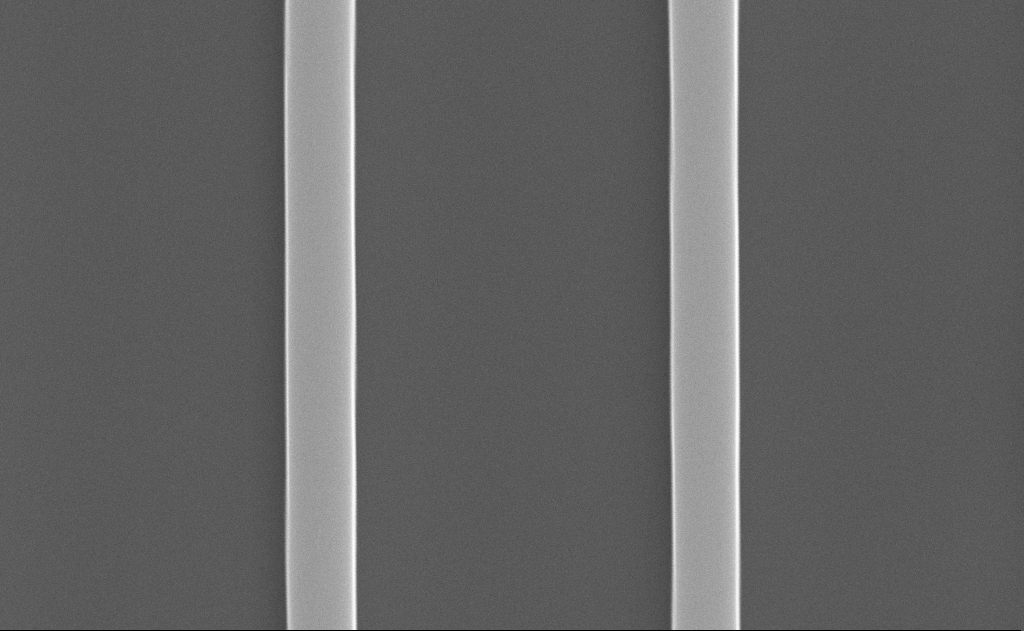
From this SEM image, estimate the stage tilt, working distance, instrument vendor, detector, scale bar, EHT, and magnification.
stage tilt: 50°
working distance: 12 mm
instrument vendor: Zeiss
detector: SE2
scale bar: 2000 nm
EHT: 10 kV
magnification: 35.27 K X